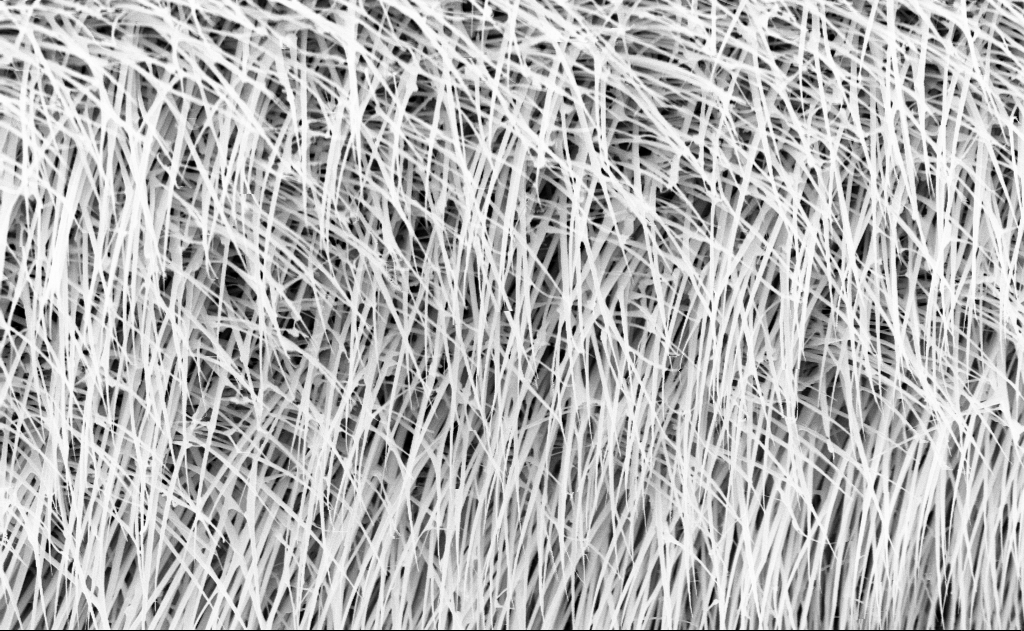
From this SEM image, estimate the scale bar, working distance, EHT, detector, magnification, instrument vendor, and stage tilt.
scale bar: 1000 nm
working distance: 13 mm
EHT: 10 kV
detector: InLens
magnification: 20 K X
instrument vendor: Zeiss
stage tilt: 0°